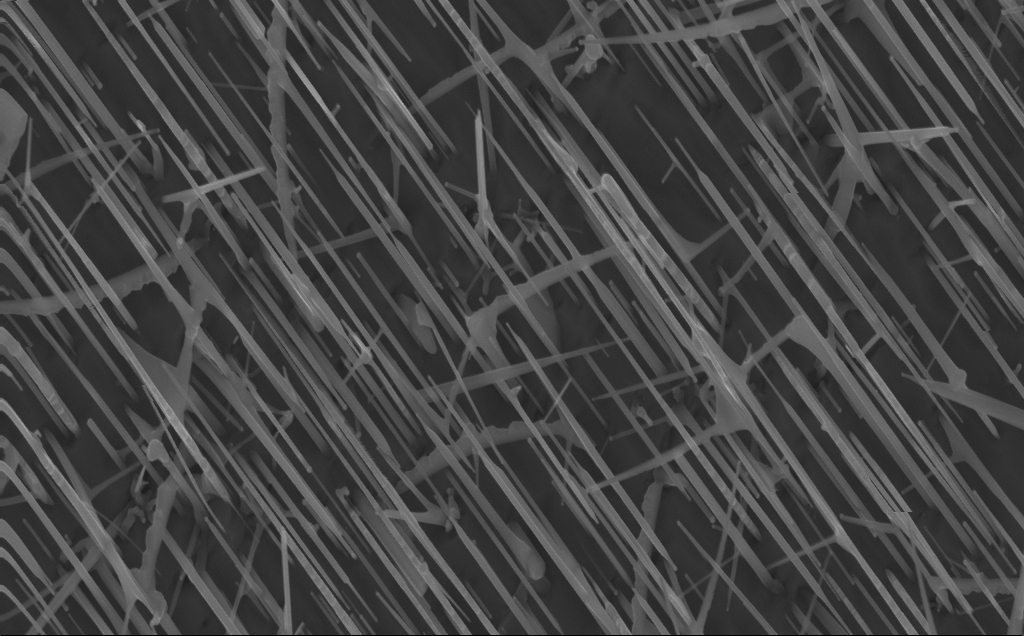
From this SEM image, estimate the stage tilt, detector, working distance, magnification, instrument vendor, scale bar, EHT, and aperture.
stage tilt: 0°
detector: InLens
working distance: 4 mm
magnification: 40 K X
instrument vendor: Zeiss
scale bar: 1000 nm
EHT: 10 kV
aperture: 30 µm